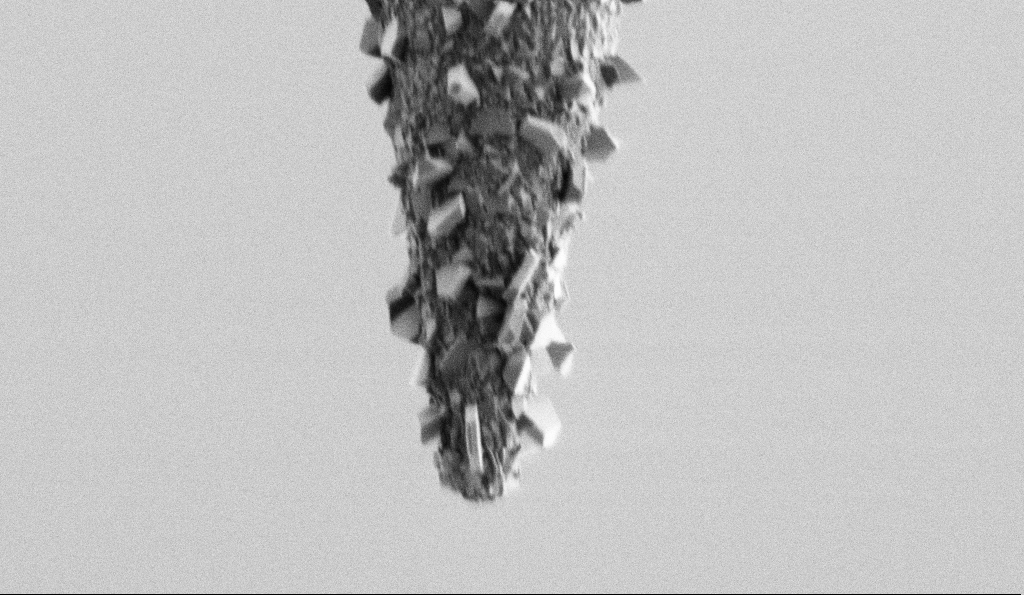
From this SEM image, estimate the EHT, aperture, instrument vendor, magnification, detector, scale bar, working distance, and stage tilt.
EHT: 1 kV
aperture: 30 µm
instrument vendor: Zeiss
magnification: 25 K X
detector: SE2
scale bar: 2000 nm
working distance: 6.7 mm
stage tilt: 0°